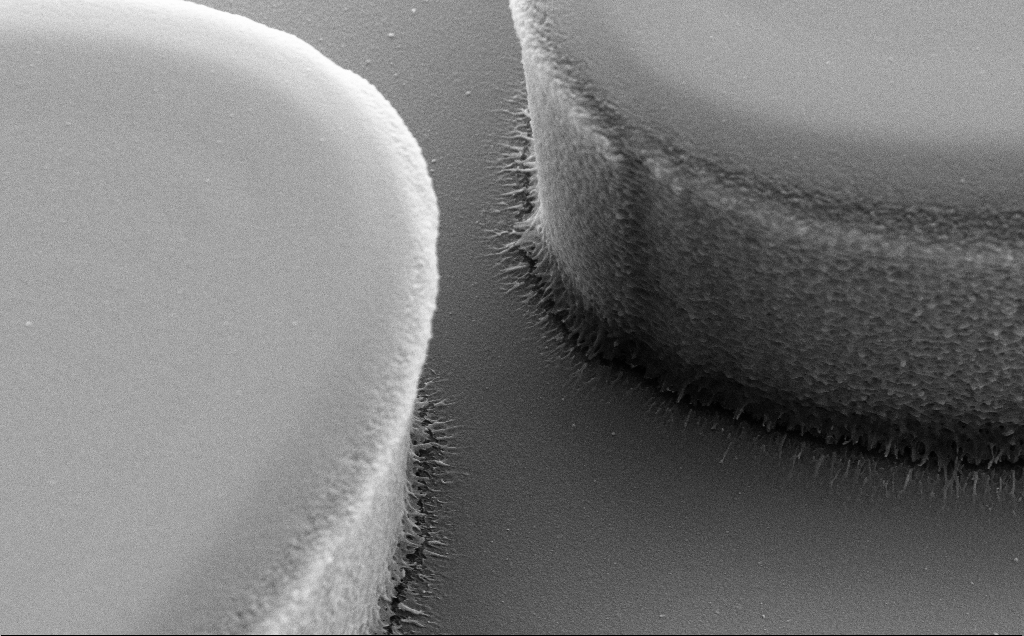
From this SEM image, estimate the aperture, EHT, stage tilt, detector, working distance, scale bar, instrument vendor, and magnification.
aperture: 30 µm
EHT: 5 kV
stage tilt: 30°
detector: SE2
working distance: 8 mm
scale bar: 2000 nm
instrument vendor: Zeiss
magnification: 18.18 K X